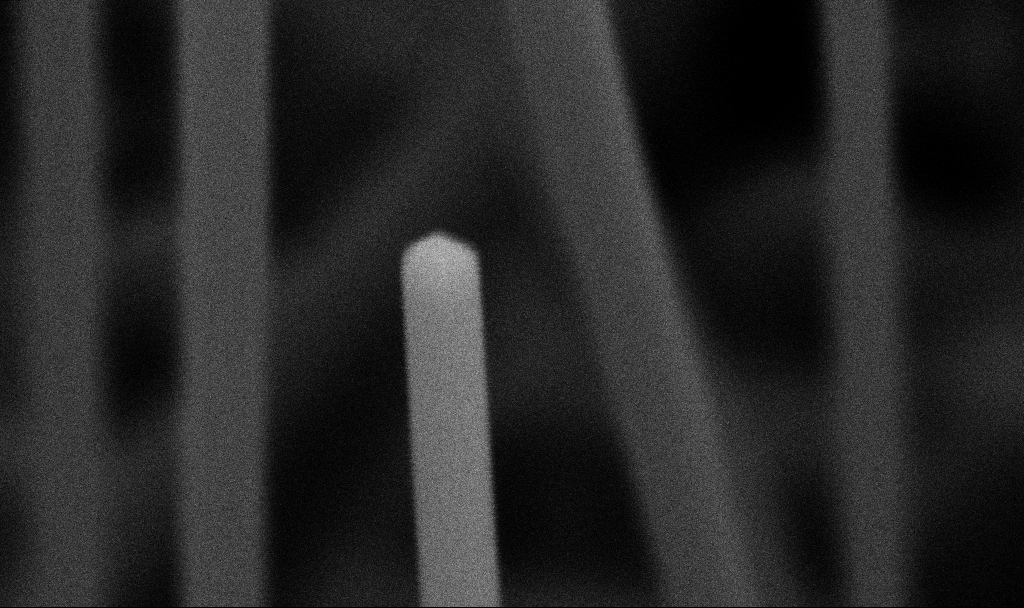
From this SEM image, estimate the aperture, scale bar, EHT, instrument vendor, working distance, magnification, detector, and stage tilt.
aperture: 30 µm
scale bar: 100 nm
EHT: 5 kV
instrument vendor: Zeiss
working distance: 7.2 mm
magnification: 283.44 K X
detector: SE2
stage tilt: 45°